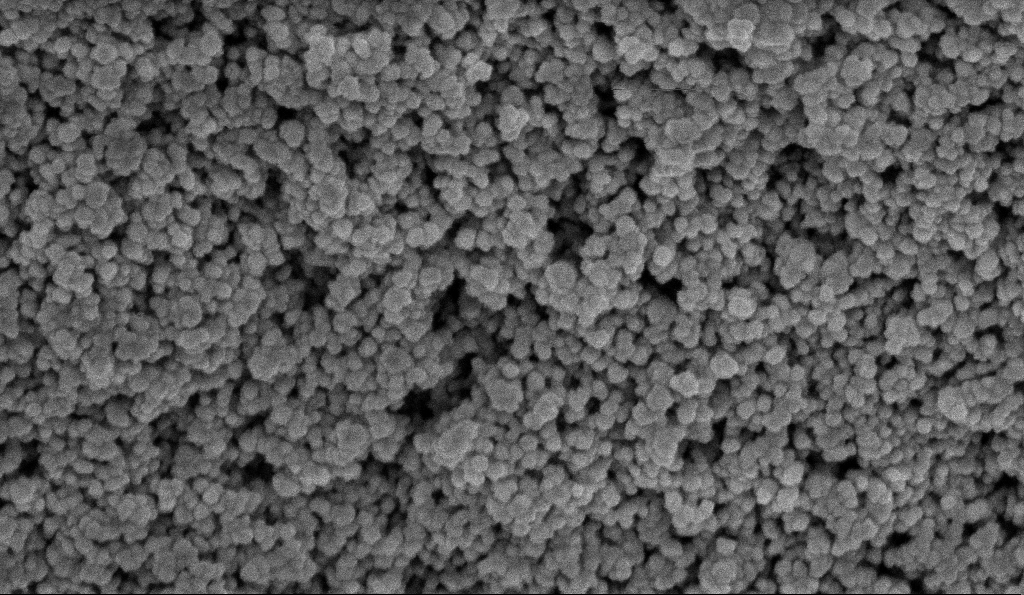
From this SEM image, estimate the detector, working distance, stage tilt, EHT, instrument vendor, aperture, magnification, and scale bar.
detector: InLens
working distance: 5.9 mm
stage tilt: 0°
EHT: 5 kV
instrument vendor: Zeiss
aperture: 30 µm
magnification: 135 K X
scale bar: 200 nm